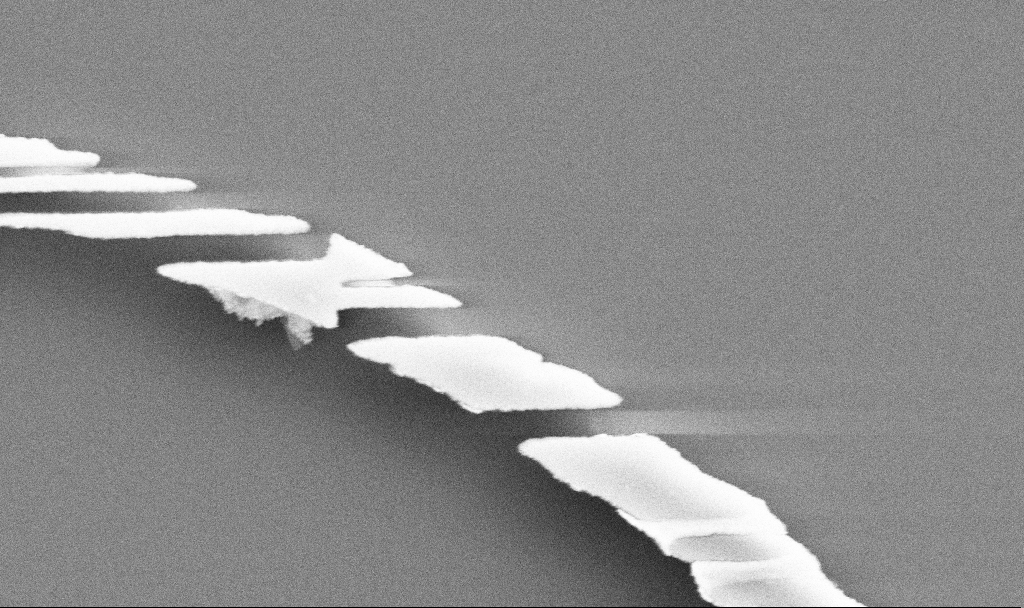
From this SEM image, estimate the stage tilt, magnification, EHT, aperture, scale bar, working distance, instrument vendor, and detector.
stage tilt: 0°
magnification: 66.89 K X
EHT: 5 kV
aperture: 30 µm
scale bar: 1000 nm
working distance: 7.5 mm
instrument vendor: Zeiss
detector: SE2